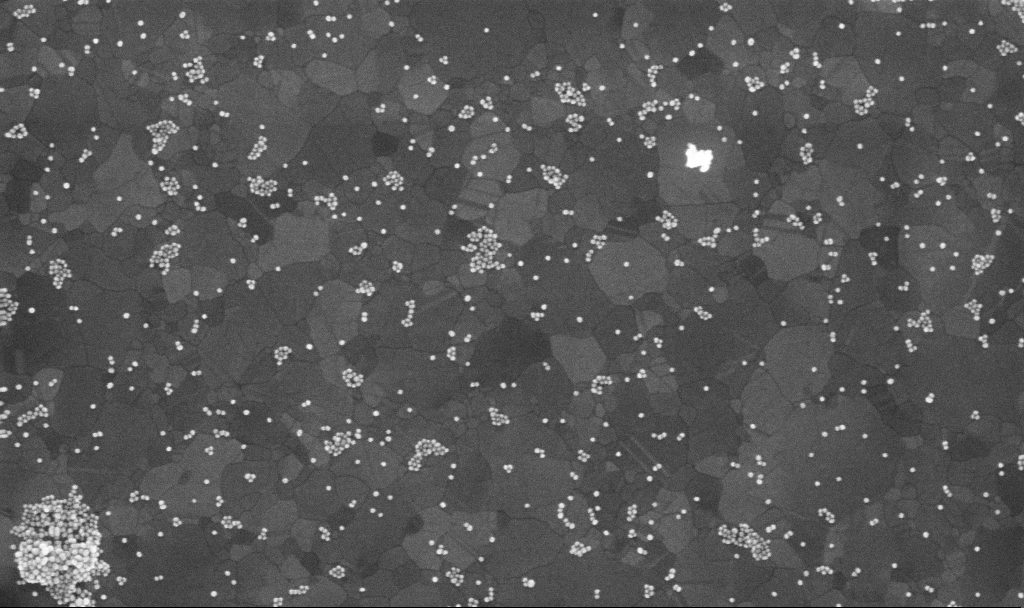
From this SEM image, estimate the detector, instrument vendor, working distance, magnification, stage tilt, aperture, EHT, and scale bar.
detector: InLens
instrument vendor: Zeiss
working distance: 3.8 mm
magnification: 100 K X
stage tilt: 0°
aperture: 30 µm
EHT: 10 kV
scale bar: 200 nm